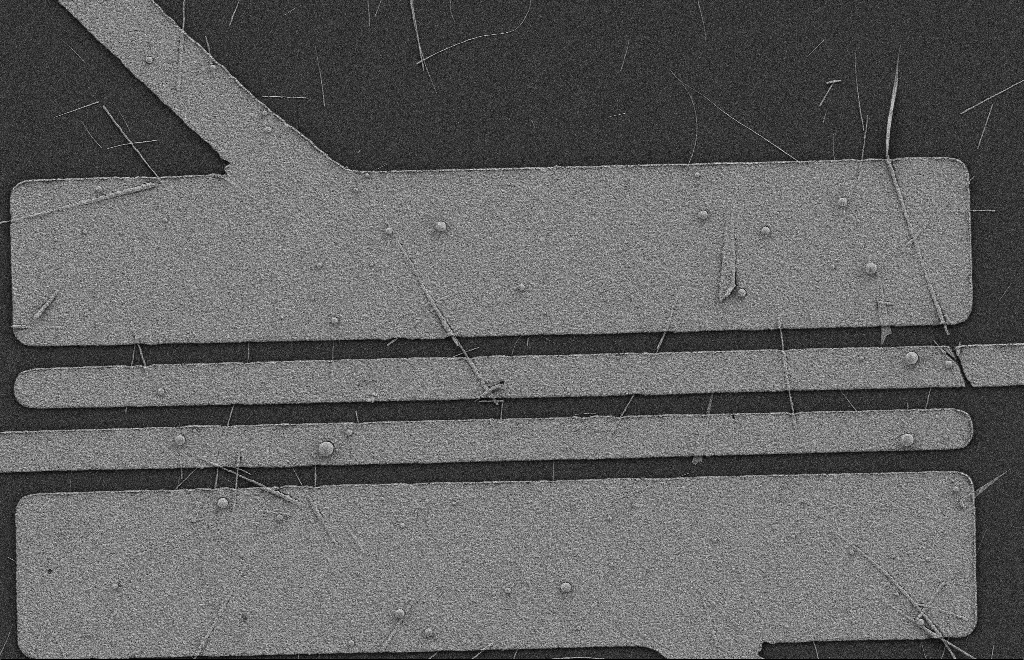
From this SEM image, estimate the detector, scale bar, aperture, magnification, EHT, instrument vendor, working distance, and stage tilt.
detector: SE2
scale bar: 2000 nm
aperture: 20 µm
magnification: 5.75 K X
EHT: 2 kV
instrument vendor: Zeiss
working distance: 8 mm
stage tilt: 0°